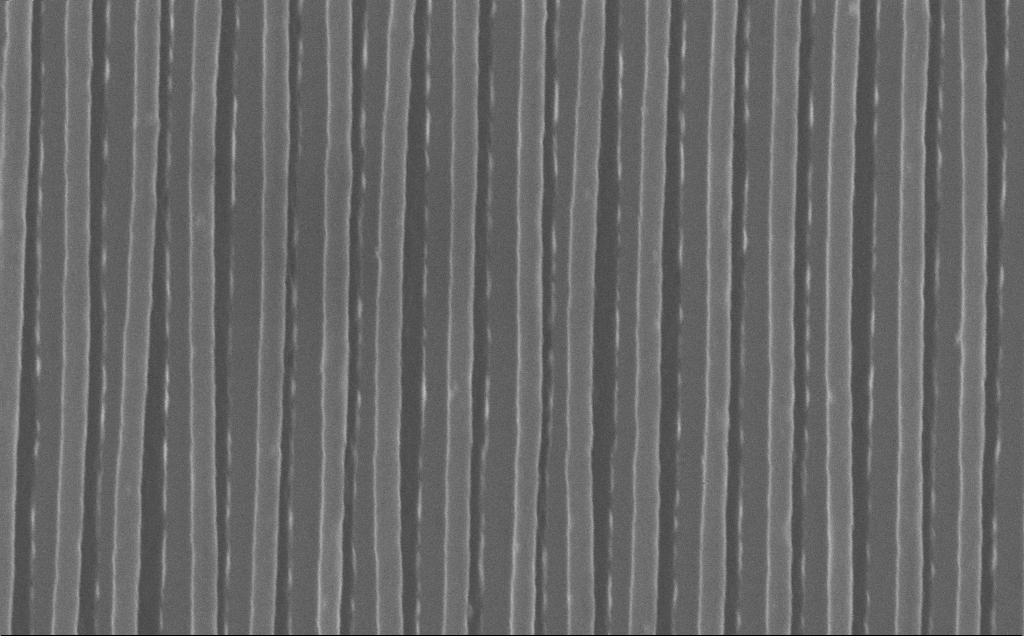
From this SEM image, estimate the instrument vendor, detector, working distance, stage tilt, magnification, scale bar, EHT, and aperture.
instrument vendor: Zeiss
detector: InLens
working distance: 7 mm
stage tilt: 0°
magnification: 78.58 K X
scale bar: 200 nm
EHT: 10 kV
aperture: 30 µm